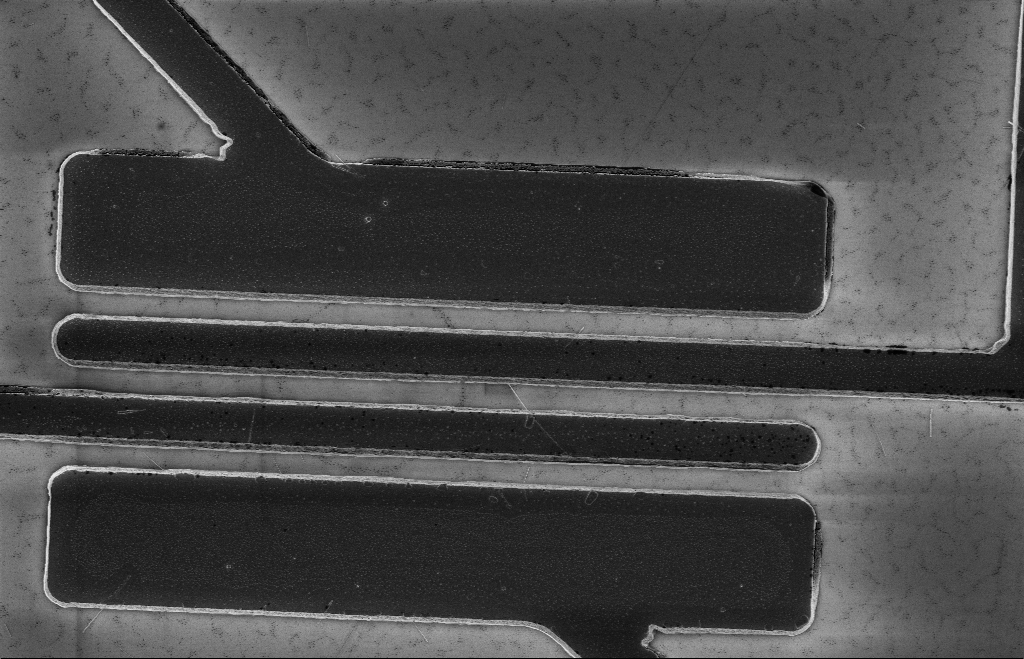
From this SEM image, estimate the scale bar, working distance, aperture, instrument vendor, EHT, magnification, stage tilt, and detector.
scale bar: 10000 nm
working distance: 12 mm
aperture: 20 µm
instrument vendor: Zeiss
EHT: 5 kV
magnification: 4.67 K X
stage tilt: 0°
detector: InLens